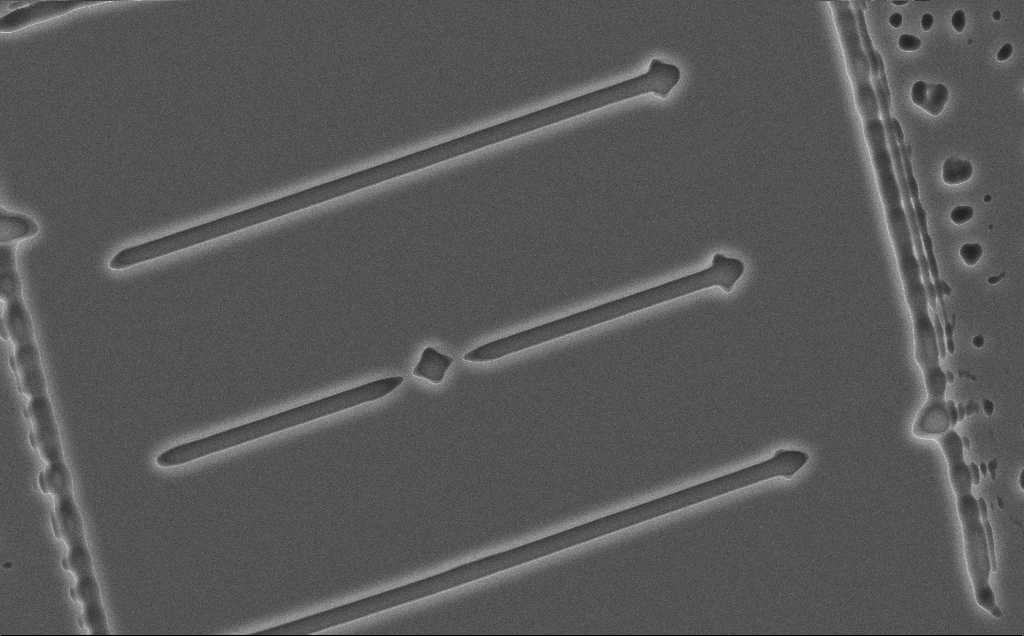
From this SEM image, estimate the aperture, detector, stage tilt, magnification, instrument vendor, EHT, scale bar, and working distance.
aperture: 30 µm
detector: SE2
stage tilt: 0°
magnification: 3.73 K X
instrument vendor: Zeiss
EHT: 10 kV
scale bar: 10000 nm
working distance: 12 mm